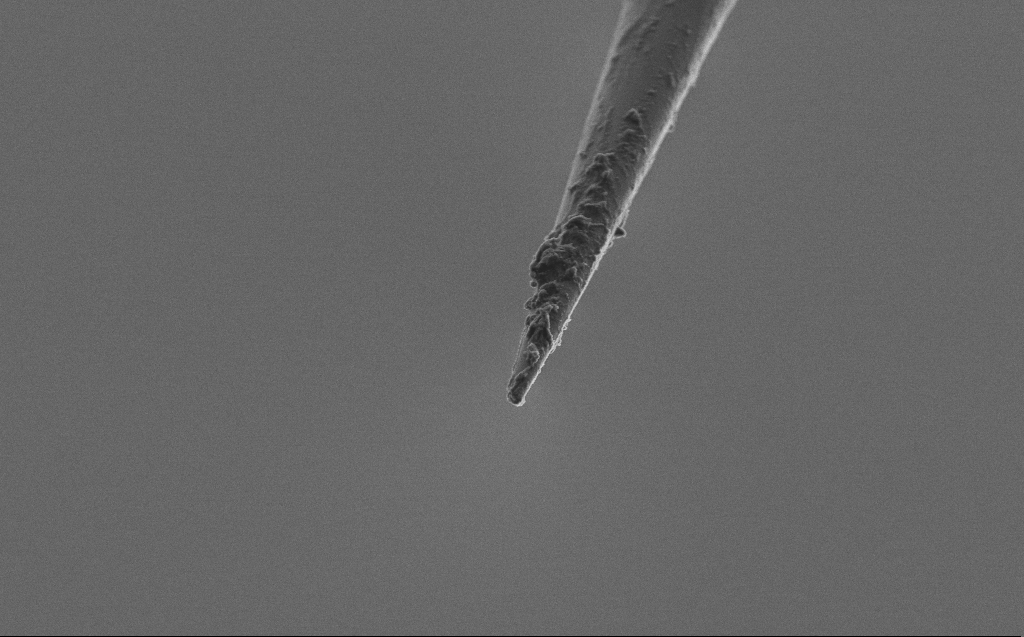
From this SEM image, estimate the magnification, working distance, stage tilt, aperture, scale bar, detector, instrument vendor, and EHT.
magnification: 10 K X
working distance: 3 mm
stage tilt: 45°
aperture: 30 µm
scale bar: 2000 nm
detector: SE2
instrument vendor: Zeiss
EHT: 2 kV